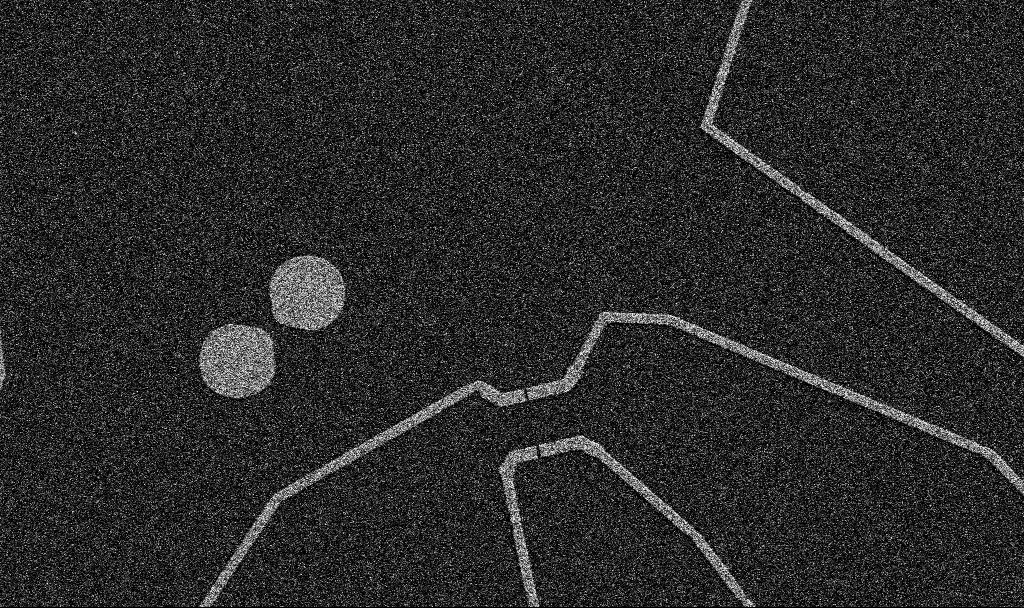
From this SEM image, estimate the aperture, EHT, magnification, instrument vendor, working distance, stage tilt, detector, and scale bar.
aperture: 30 µm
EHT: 5 kV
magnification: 5 K X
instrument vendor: Zeiss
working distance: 10.7 mm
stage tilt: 0°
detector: SE2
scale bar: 10000 nm